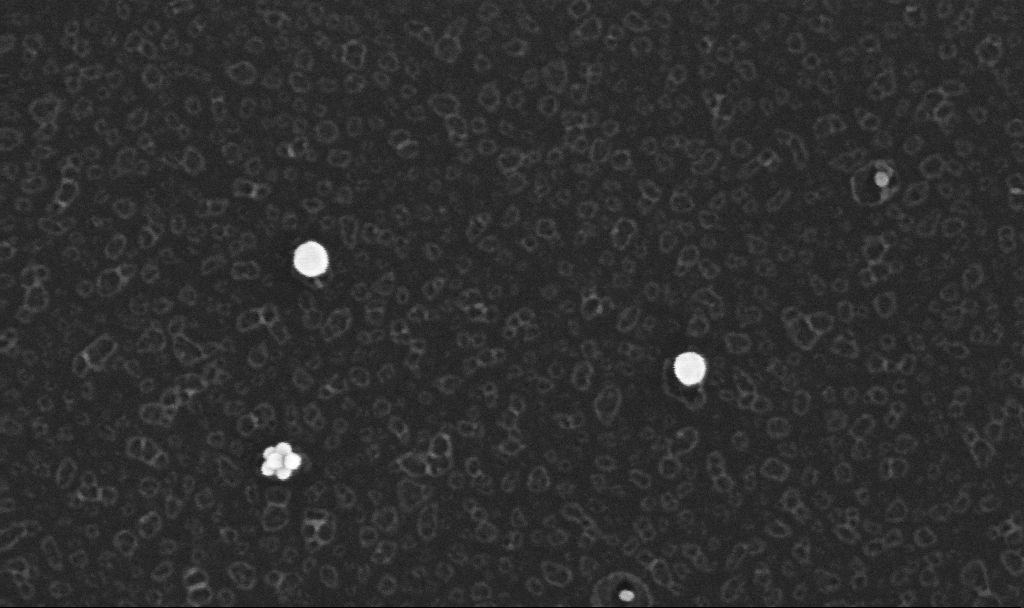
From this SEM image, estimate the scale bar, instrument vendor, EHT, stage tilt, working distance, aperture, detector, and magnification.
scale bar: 200 nm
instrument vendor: Zeiss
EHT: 10 kV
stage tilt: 0°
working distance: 3.4 mm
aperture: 30 µm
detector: InLens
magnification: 292.9 K X